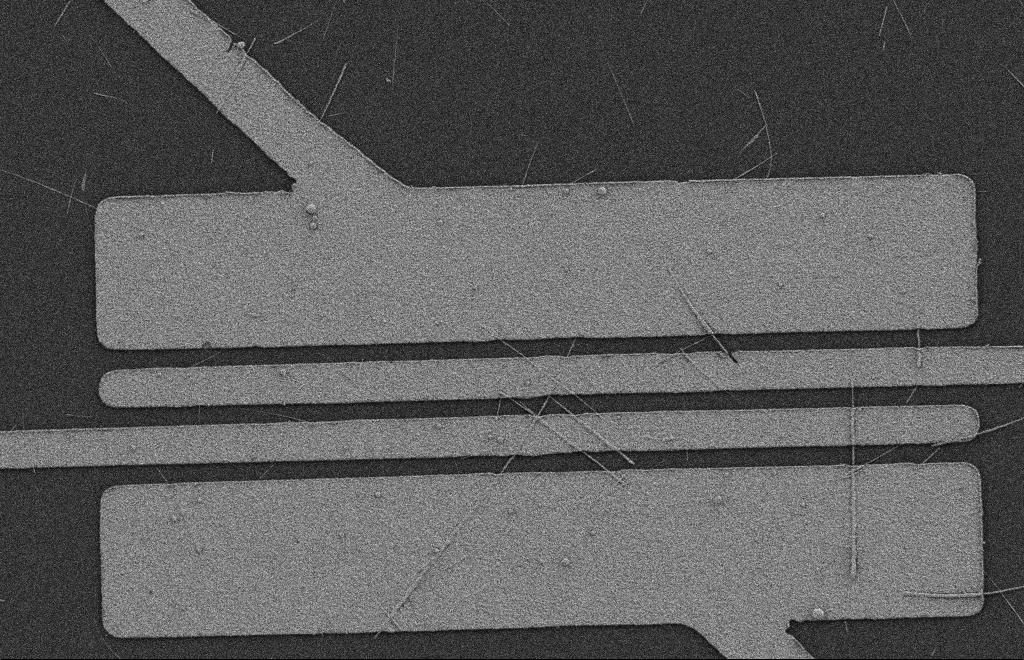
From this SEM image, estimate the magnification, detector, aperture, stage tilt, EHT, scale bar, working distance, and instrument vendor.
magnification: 5.29 K X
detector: SE2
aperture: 20 µm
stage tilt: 0°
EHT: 2 kV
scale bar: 2000 nm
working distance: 9 mm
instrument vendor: Zeiss